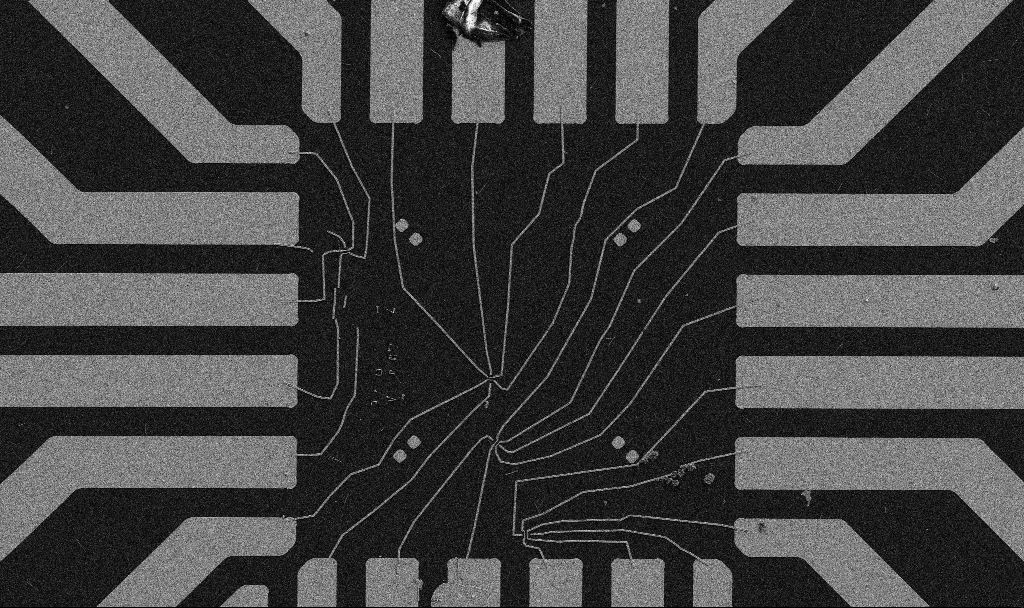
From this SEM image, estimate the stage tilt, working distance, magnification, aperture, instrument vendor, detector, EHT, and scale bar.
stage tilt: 0°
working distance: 10.8 mm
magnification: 1 K X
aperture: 30 µm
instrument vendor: Zeiss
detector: SE2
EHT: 5 kV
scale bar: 20000 nm